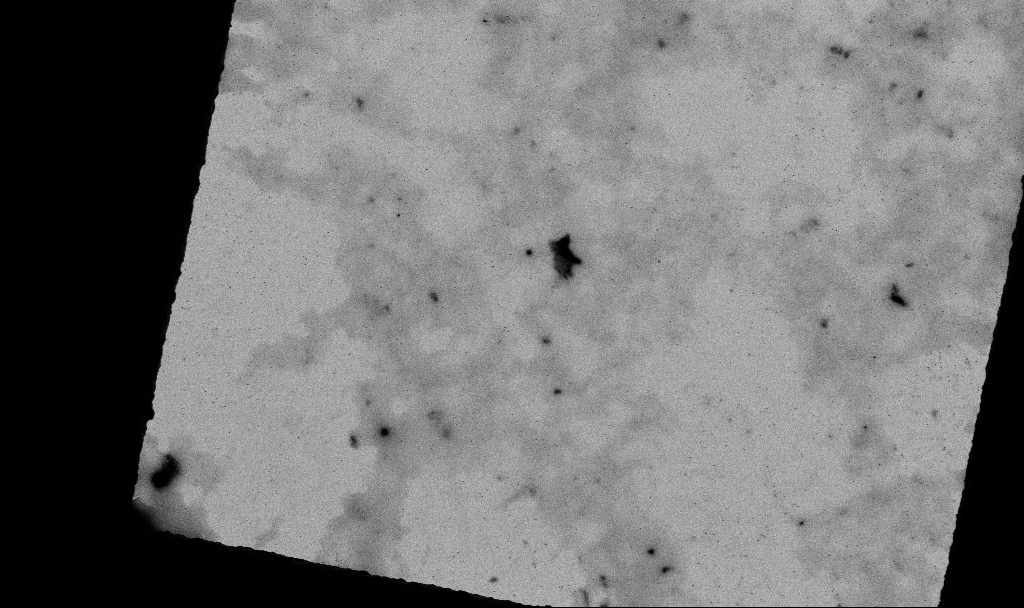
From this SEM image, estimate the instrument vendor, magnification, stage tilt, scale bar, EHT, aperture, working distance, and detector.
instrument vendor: Zeiss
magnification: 3.3 K X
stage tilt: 0°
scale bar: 10000 nm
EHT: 5 kV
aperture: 30 µm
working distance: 4.7 mm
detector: SE2